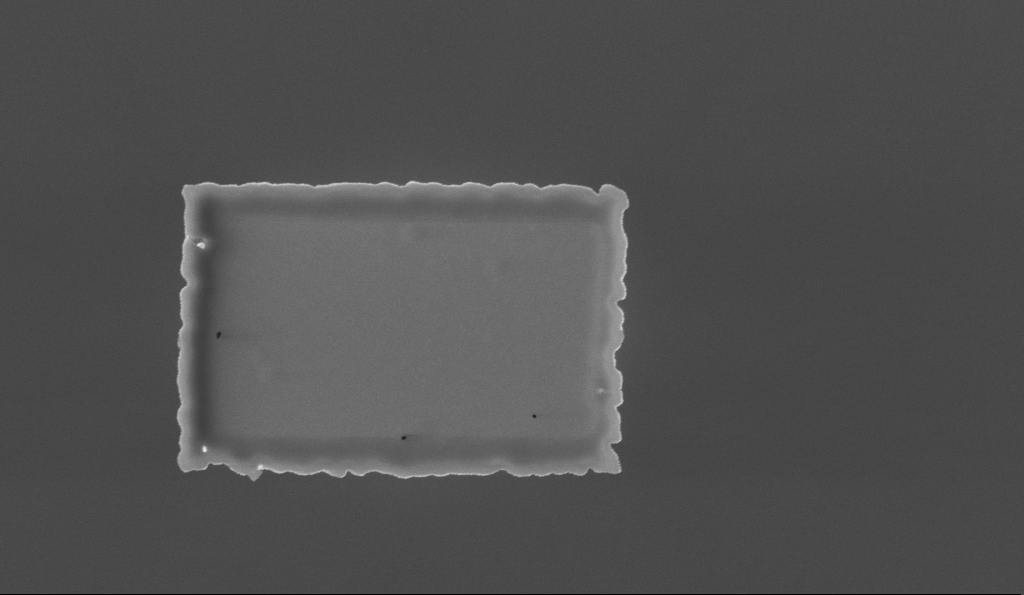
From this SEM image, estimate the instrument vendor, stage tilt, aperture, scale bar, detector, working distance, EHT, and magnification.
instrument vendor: Zeiss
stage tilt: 0.7°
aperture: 30 µm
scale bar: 1000 nm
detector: InLens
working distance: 5.7 mm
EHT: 5 kV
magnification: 36.84 K X